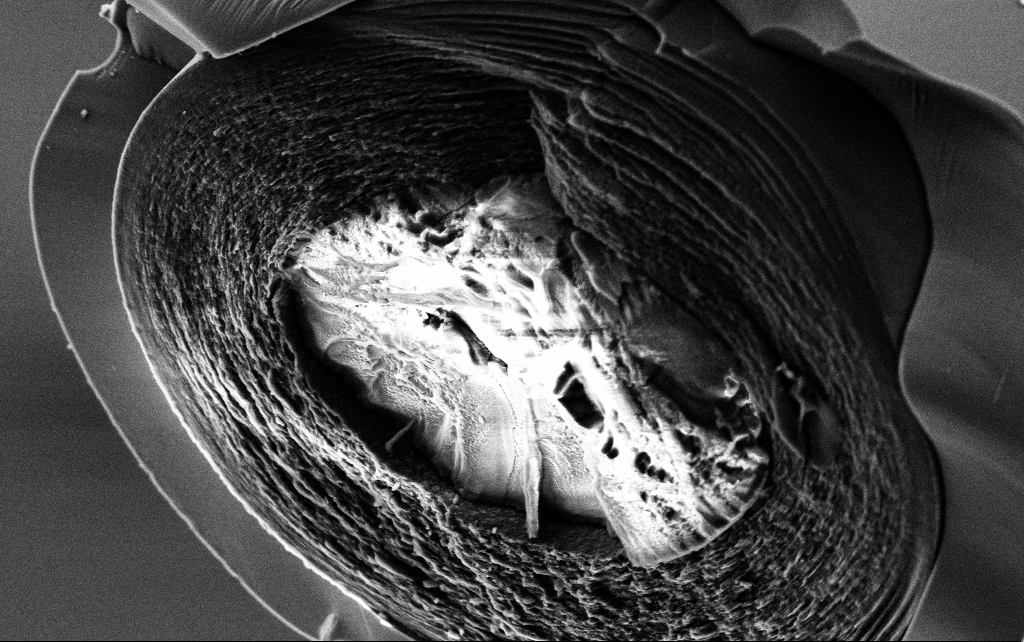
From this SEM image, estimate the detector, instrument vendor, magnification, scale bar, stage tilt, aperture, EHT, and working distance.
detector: SE2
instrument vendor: Zeiss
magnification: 15 K X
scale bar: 2000 nm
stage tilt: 45°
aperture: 30 µm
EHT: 3 kV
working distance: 7.8 mm